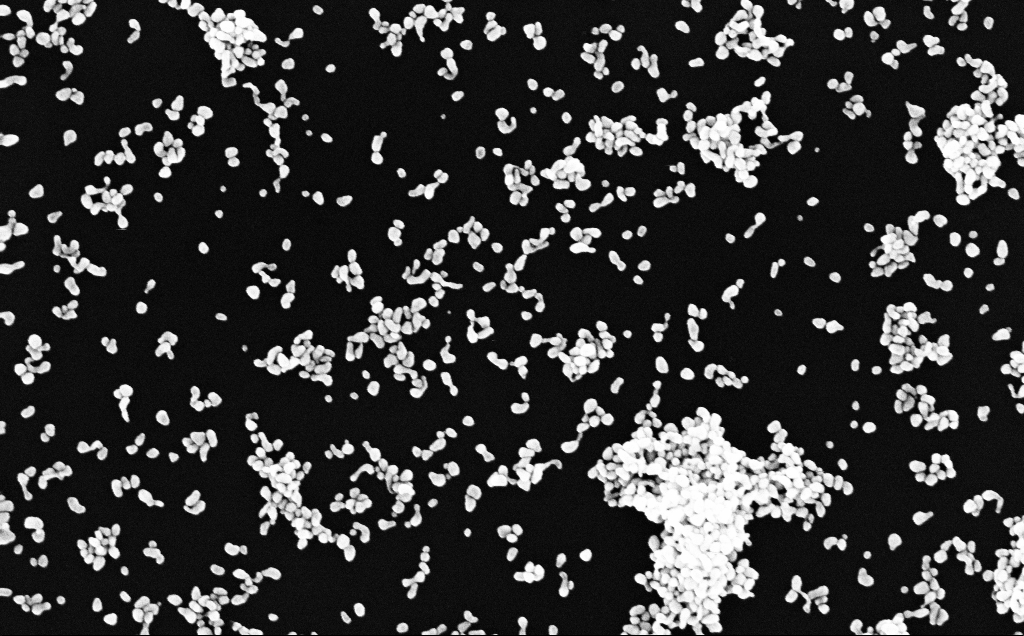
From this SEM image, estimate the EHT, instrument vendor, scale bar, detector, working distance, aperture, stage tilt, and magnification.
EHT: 10 kV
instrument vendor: Zeiss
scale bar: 200 nm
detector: InLens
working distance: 3.1 mm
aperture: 30 µm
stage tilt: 0°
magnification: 100 K X